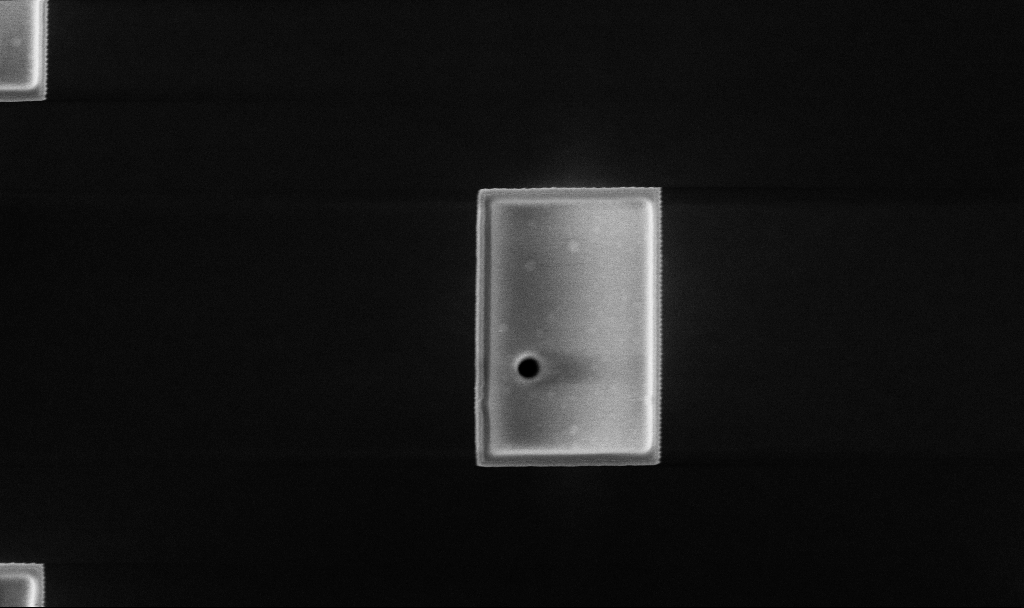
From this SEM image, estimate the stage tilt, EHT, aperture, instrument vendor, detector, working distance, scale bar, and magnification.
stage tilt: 0.2°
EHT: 5 kV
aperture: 30 µm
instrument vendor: Zeiss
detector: InLens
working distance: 3.9 mm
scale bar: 1000 nm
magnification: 23.23 K X